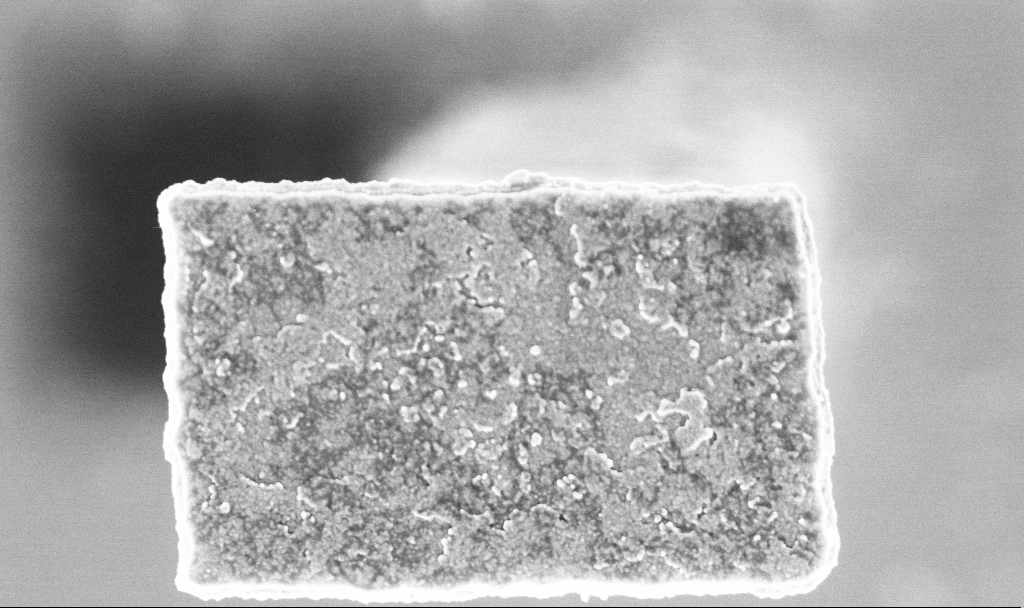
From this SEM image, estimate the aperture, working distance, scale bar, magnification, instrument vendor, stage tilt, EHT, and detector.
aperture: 30 µm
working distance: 3.2 mm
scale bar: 200 nm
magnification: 82.89 K X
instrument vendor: Zeiss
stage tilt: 0°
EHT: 3 kV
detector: InLens